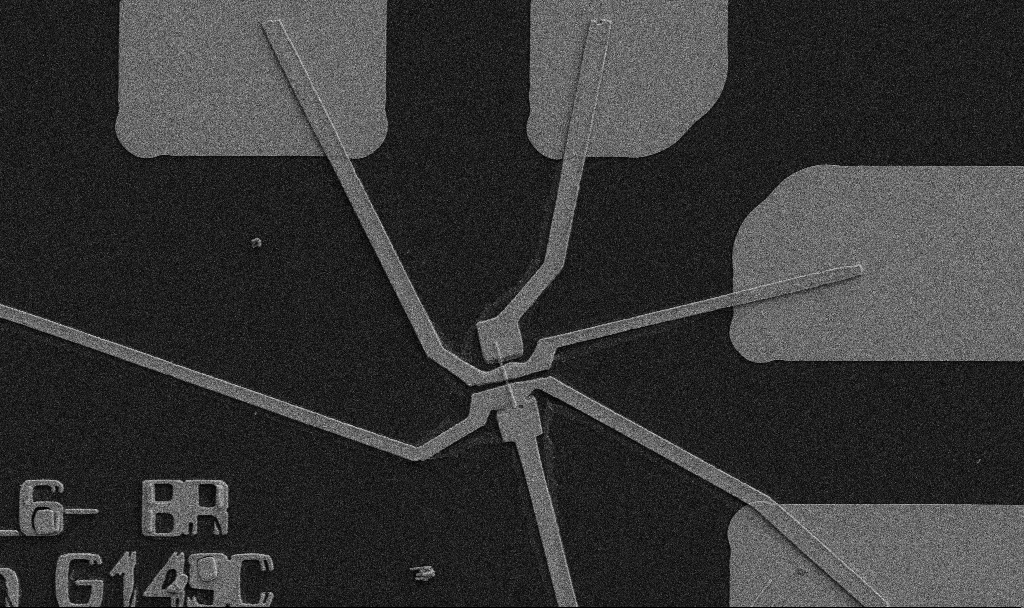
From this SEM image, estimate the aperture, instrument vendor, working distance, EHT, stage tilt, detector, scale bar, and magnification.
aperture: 30 µm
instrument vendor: Zeiss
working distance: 10.7 mm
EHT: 5 kV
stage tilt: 0°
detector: SE2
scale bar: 10000 nm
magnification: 5 K X